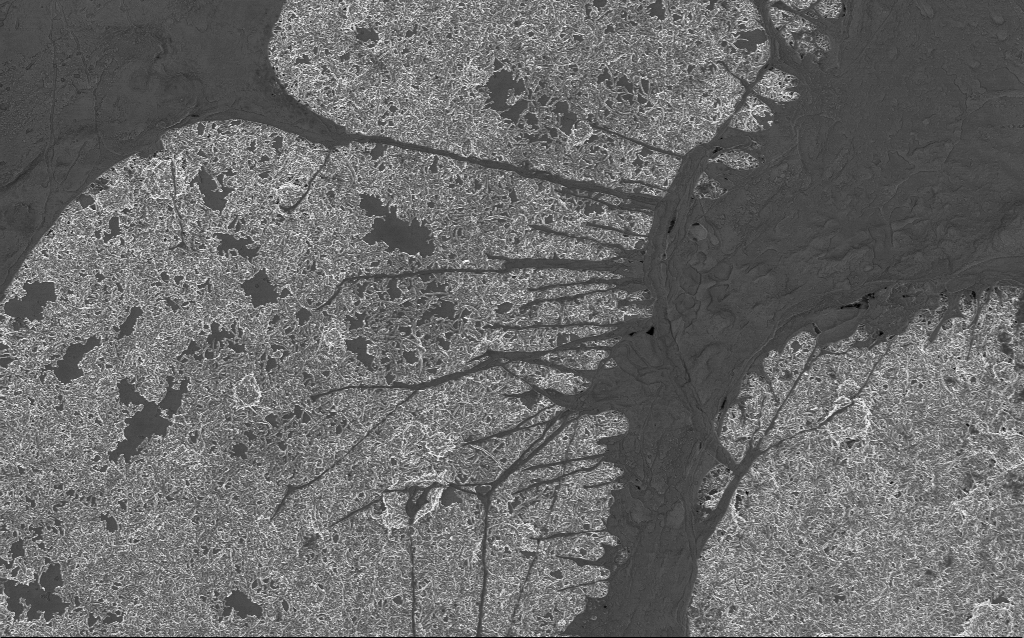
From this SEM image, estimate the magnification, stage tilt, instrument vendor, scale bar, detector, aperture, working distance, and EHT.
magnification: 10 K X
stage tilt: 0°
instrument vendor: Zeiss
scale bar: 2000 nm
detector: InLens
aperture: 30 µm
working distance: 6 mm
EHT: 1 kV